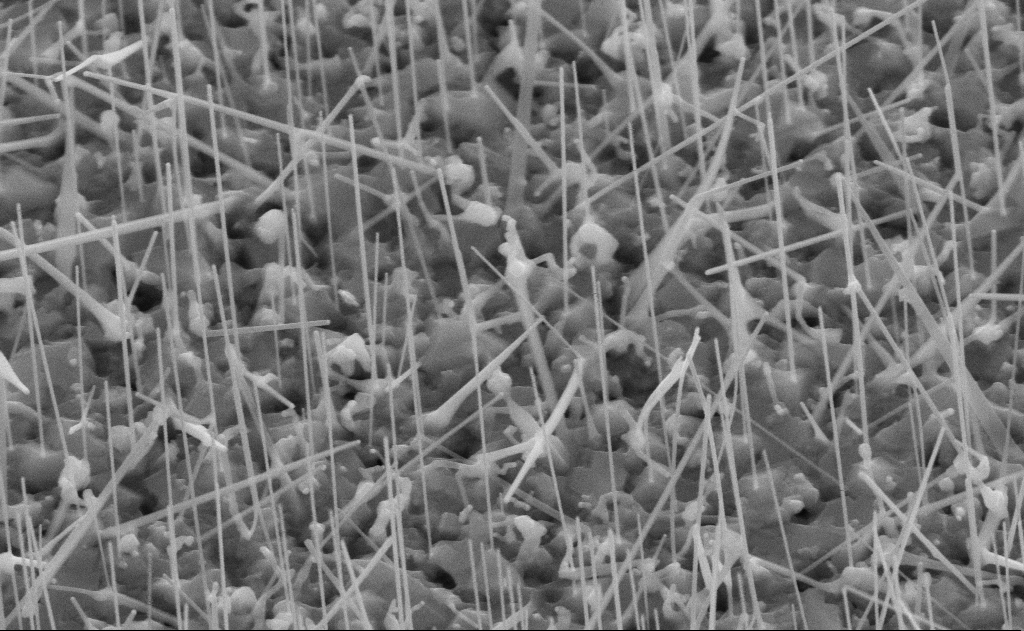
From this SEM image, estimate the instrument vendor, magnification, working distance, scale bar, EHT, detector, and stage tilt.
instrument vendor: Zeiss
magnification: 60 K X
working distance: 11 mm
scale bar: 1000 nm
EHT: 10 kV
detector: SE2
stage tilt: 45°